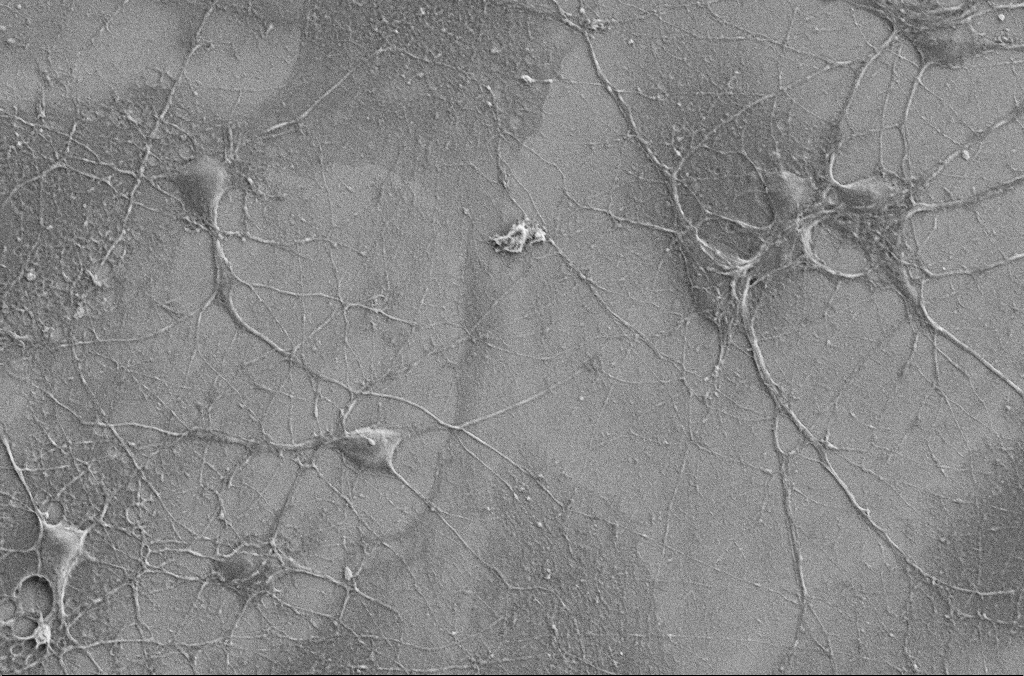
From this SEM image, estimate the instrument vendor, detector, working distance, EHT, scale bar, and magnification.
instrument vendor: Zeiss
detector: SE2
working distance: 5.9 mm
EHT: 5 kV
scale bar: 20000 nm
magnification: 2 K X